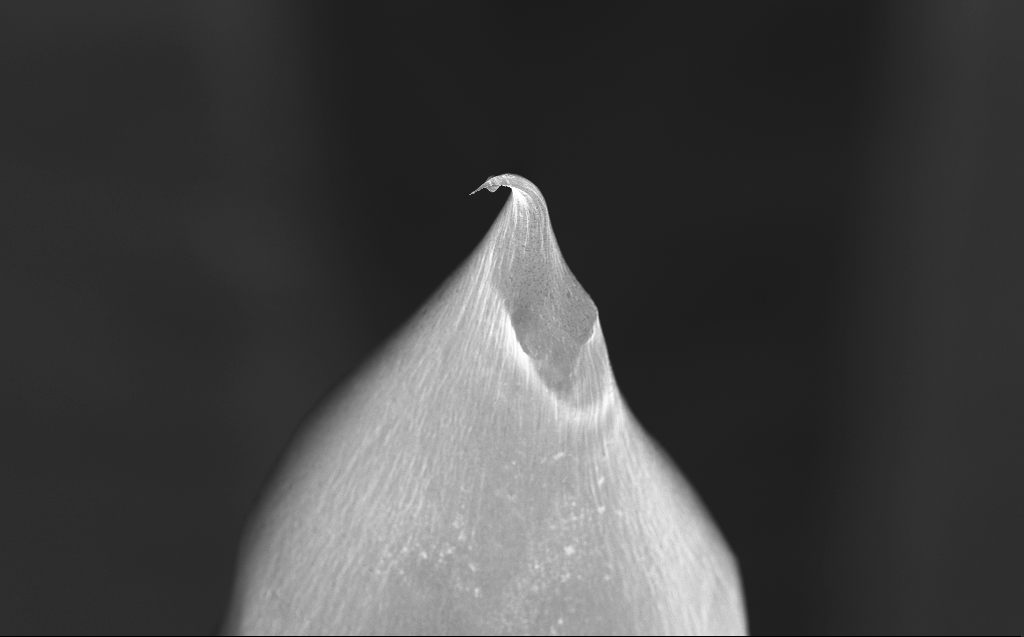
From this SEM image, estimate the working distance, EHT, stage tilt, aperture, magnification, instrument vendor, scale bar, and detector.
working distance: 3 mm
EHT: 10 kV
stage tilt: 40°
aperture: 30 µm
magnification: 0.742 K X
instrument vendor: Zeiss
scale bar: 20000 nm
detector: InLens